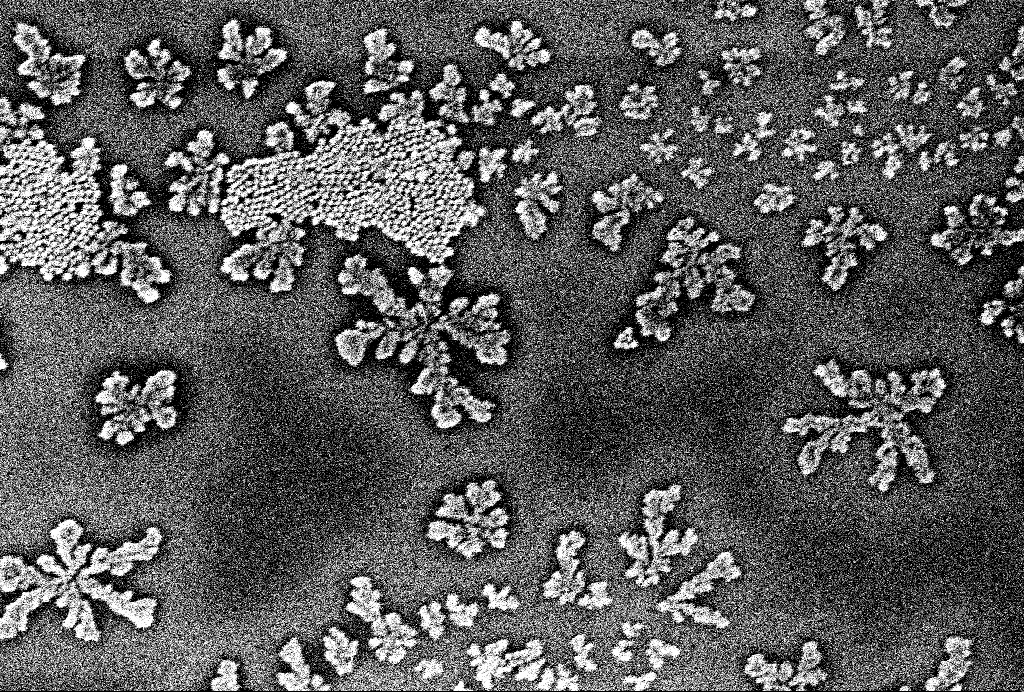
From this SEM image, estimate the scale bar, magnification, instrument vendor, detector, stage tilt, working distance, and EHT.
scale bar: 100 nm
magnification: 119.11 K X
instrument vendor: Zeiss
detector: InLens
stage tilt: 0°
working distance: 3.1 mm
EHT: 1 kV